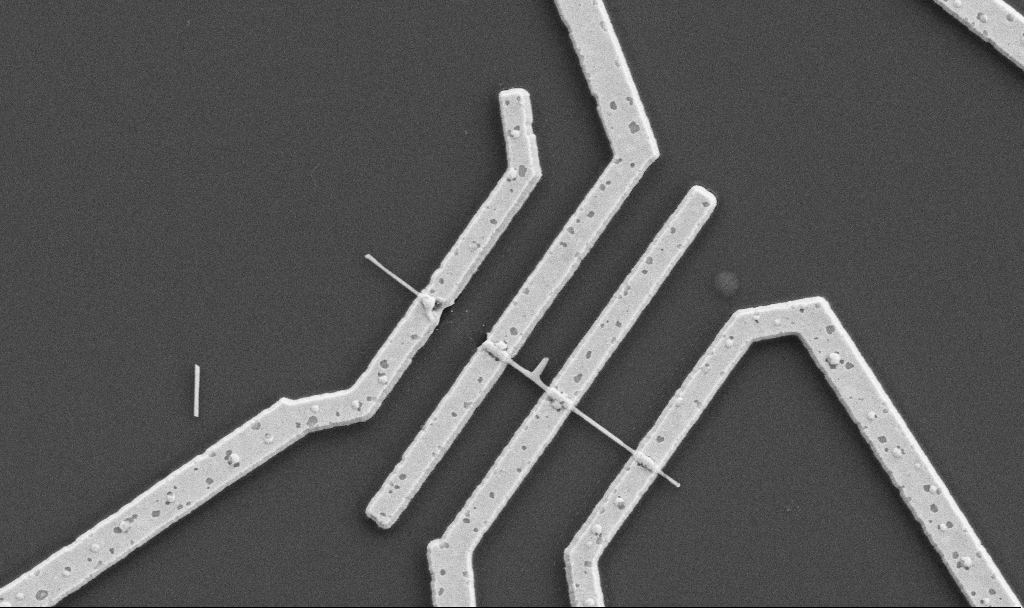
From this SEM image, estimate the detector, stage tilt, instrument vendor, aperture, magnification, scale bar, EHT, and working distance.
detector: SE2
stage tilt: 0°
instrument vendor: Zeiss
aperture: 30 µm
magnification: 20.75 K X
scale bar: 1000 nm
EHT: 5 kV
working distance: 10.6 mm